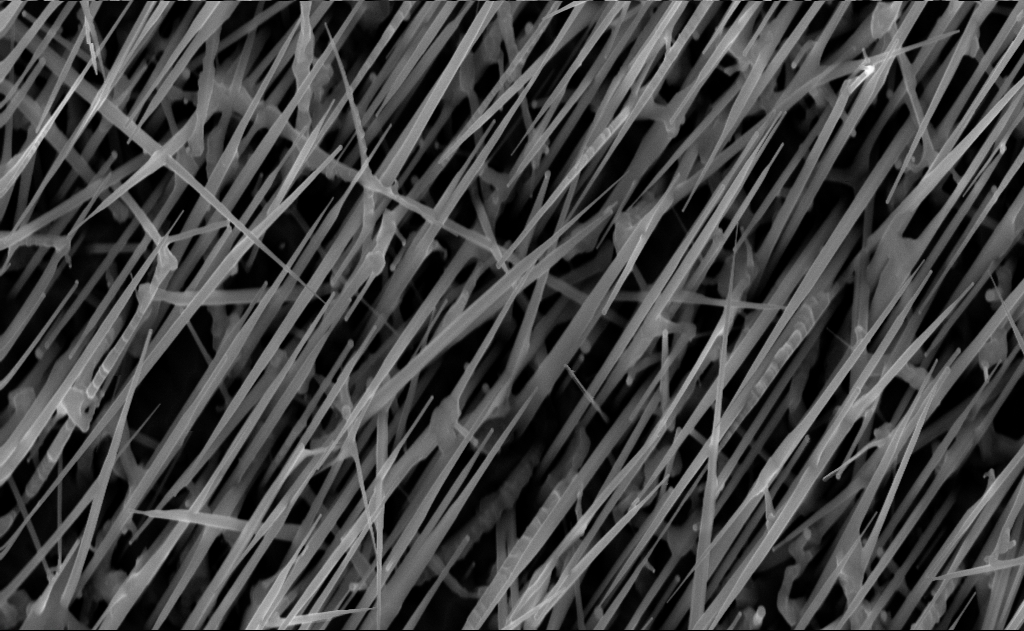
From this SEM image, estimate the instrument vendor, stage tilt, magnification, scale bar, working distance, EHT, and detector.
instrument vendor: Zeiss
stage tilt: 0°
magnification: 40 K X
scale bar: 1000 nm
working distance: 7 mm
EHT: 10 kV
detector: InLens